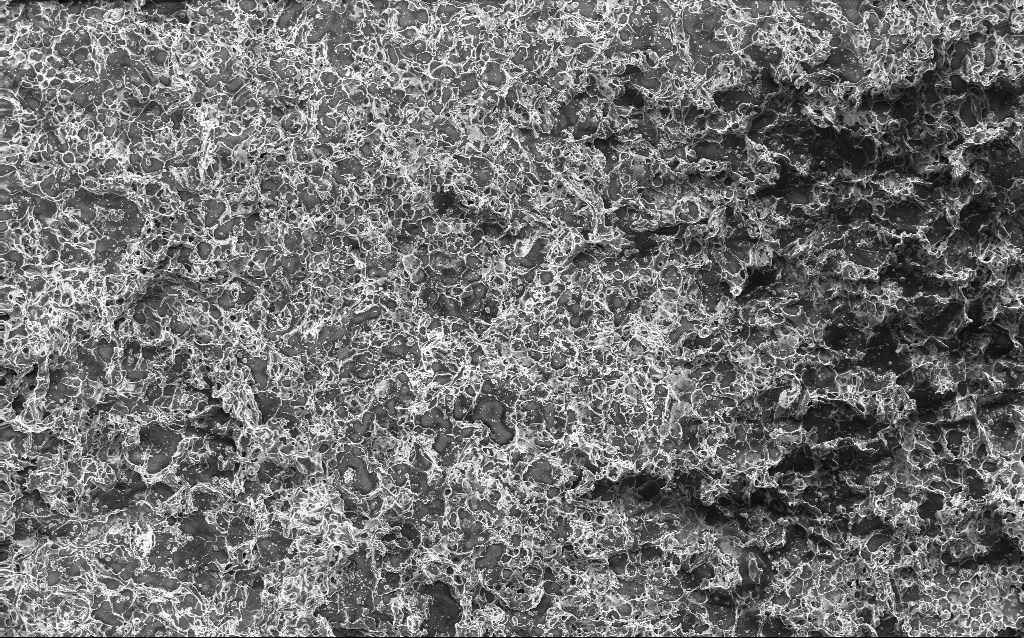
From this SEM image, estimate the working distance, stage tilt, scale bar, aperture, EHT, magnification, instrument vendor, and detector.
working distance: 2.7 mm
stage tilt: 0°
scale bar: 100000 nm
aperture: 30 µm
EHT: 10 kV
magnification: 0.235 K X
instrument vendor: Zeiss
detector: InLens